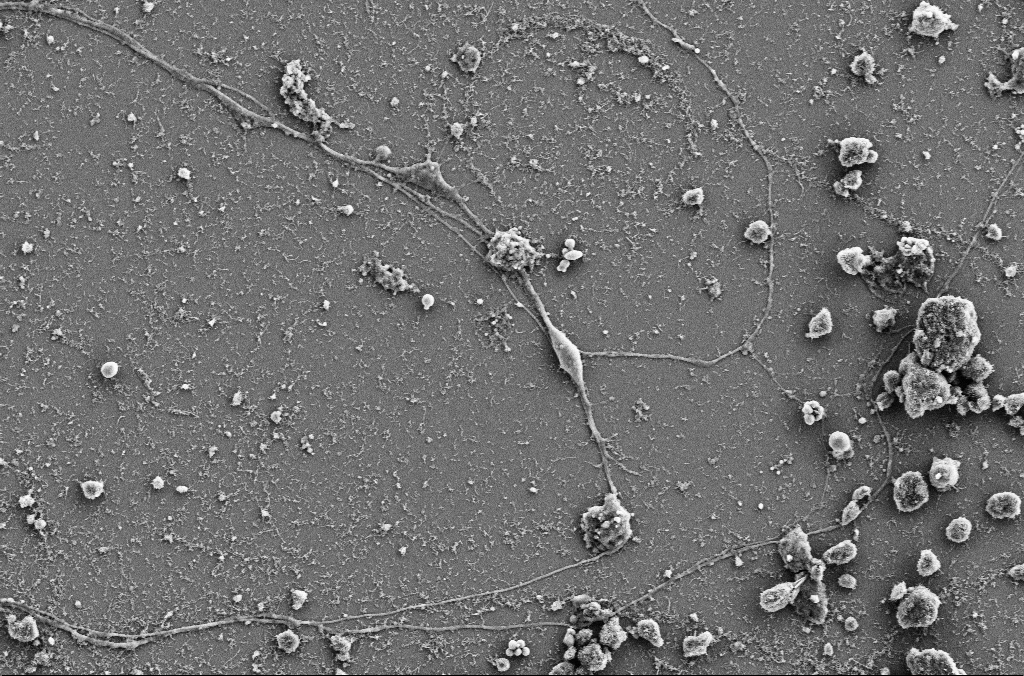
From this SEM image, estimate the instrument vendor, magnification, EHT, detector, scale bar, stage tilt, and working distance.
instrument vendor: Zeiss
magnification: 4 K X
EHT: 5 kV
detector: SE2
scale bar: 10000 nm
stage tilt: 0°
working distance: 4 mm